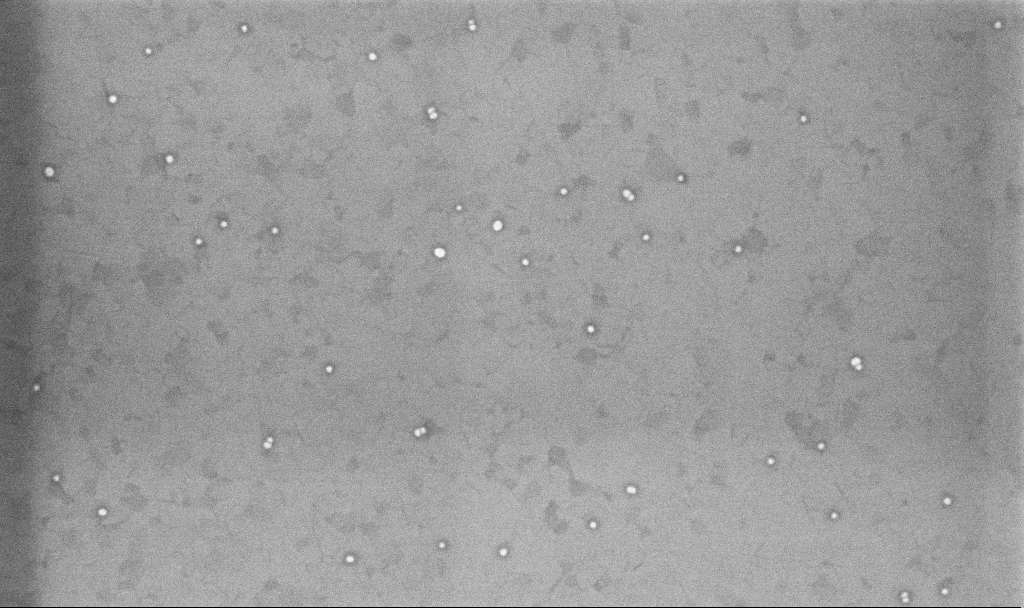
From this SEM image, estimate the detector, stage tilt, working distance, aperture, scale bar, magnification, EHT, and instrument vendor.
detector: InLens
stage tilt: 0°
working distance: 3.3 mm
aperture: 30 µm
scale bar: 200 nm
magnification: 100.38 K X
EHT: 10 kV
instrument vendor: Zeiss